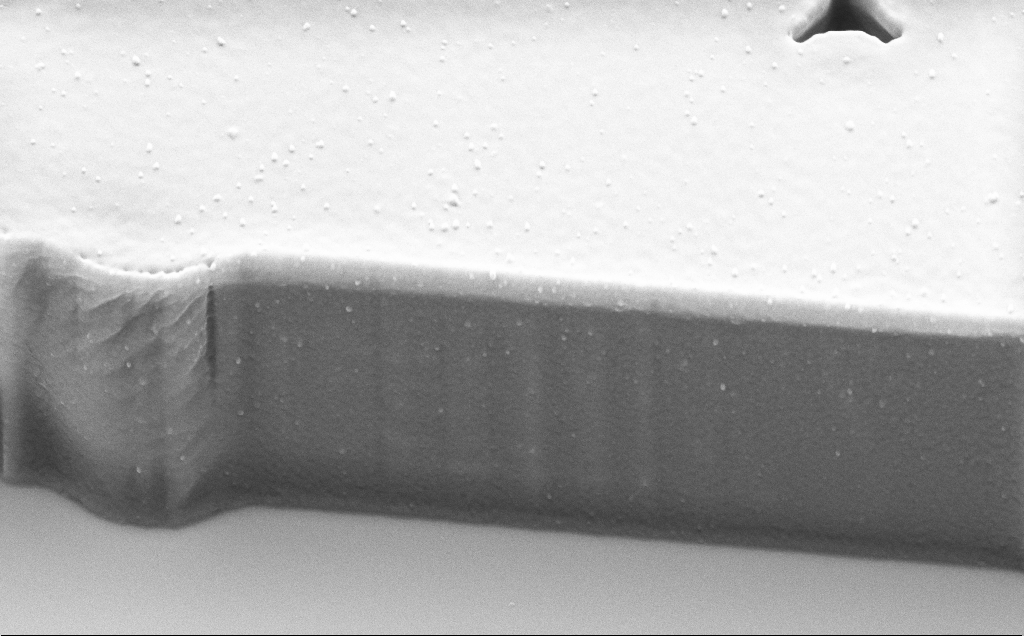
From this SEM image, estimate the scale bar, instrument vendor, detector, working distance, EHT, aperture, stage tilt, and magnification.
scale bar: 1000 nm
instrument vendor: Zeiss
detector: SE2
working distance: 9 mm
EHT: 5 kV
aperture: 30 µm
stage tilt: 45°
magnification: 24.15 K X